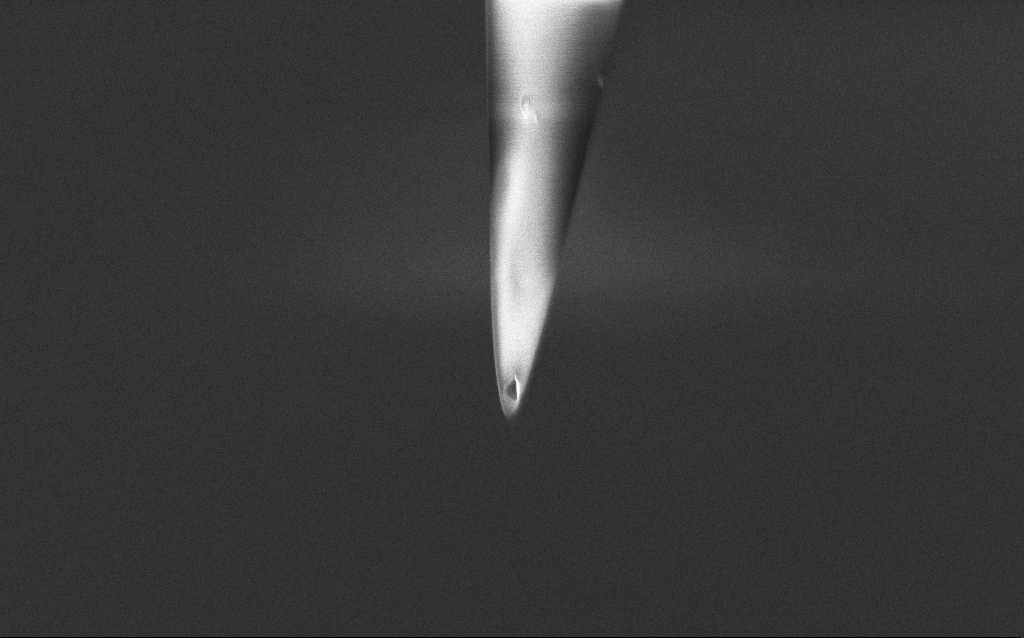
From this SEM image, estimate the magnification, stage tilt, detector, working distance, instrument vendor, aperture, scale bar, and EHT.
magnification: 27.35 K X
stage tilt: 45°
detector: InLens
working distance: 5 mm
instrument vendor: Zeiss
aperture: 30 µm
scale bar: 1000 nm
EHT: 1 kV